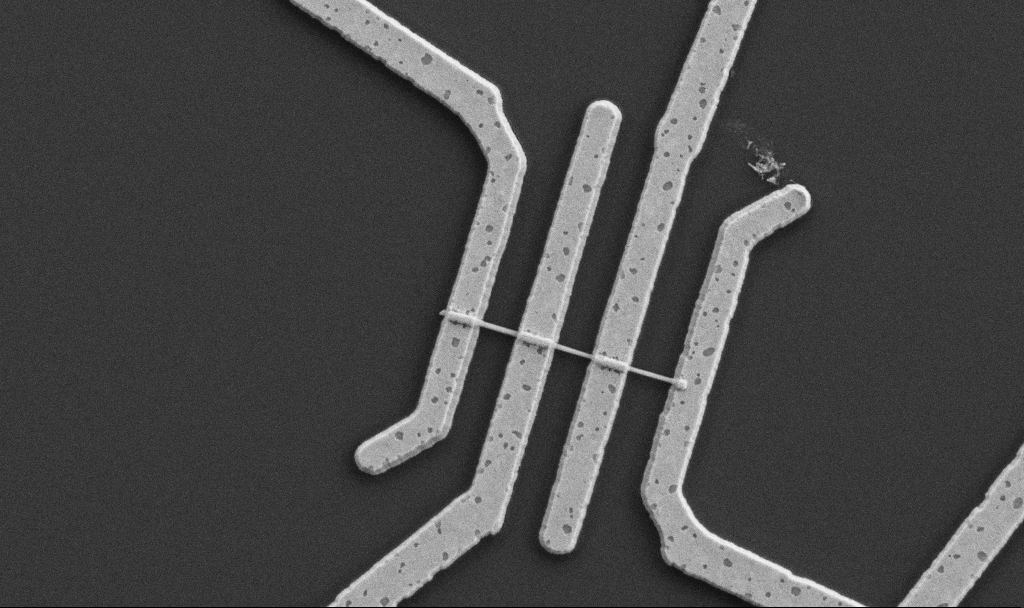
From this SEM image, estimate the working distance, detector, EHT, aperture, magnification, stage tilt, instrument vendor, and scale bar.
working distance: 10.7 mm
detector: SE2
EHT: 5 kV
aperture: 30 µm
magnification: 20 K X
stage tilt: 0°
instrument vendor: Zeiss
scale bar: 1000 nm